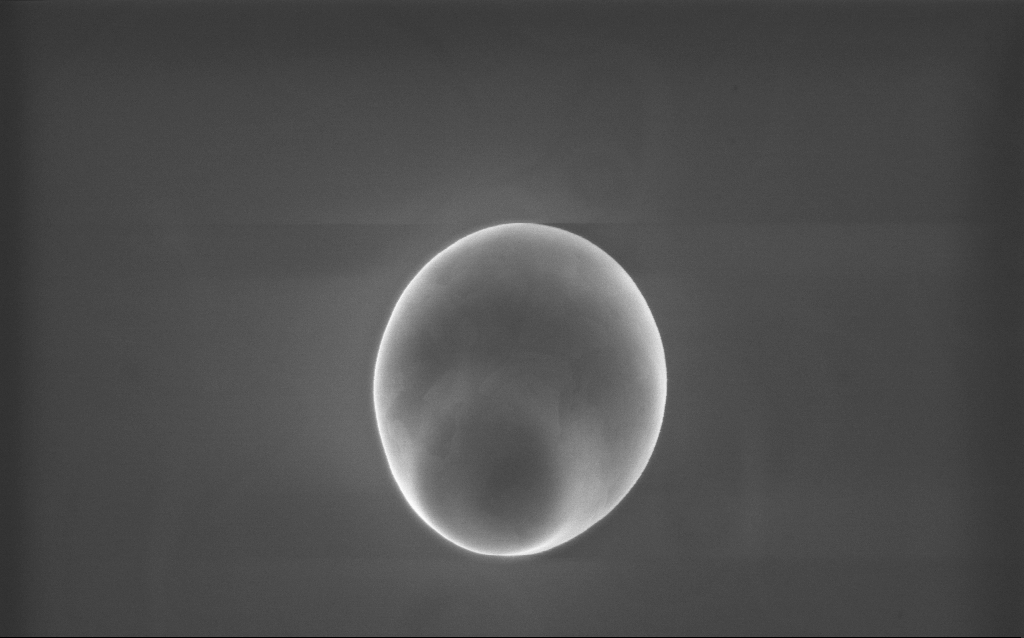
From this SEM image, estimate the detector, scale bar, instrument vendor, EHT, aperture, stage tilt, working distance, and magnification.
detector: InLens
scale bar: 1000 nm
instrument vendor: Zeiss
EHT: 10 kV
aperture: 30 µm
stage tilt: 0°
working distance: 2 mm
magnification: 42 K X